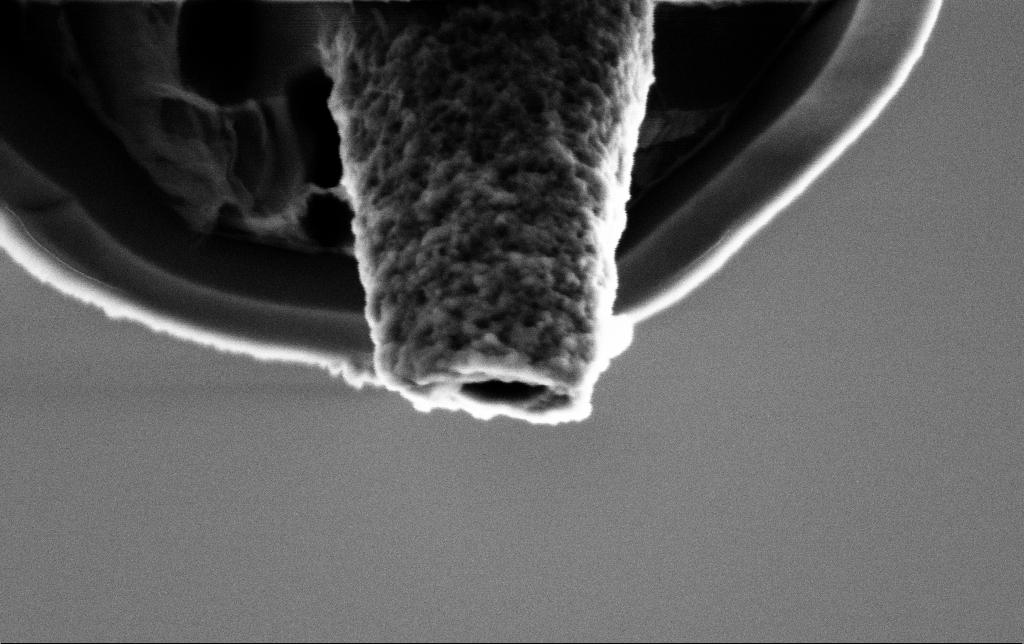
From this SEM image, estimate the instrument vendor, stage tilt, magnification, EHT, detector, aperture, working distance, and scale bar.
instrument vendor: Zeiss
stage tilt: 45°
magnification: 100 K X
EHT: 2 kV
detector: SE2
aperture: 30 µm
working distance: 7.3 mm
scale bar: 200 nm